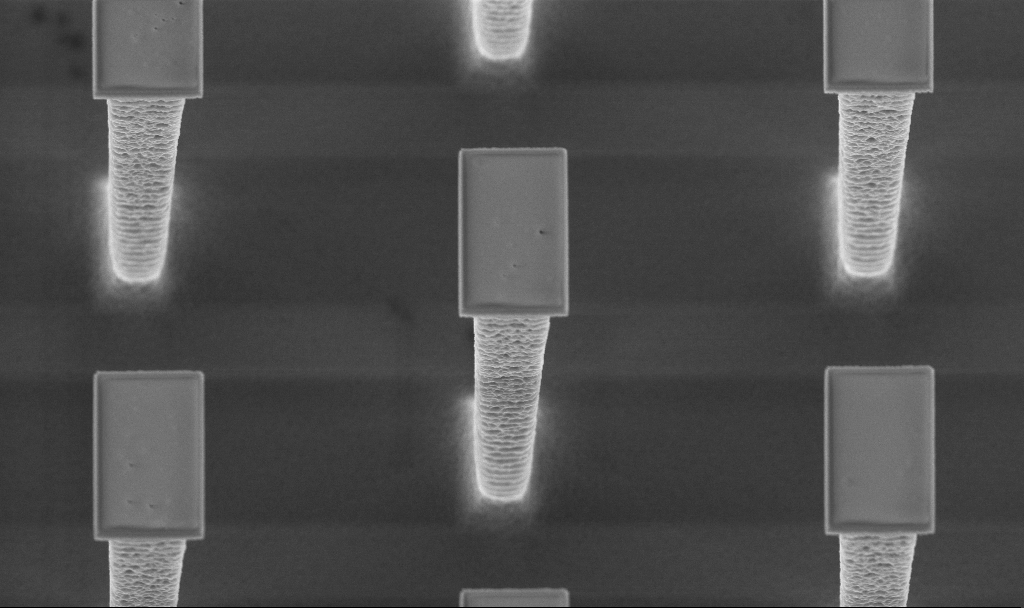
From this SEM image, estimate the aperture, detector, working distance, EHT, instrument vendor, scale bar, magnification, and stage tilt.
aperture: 30 µm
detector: InLens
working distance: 5.1 mm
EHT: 5 kV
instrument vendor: Zeiss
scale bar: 1000 nm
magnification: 13.35 K X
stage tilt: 20°